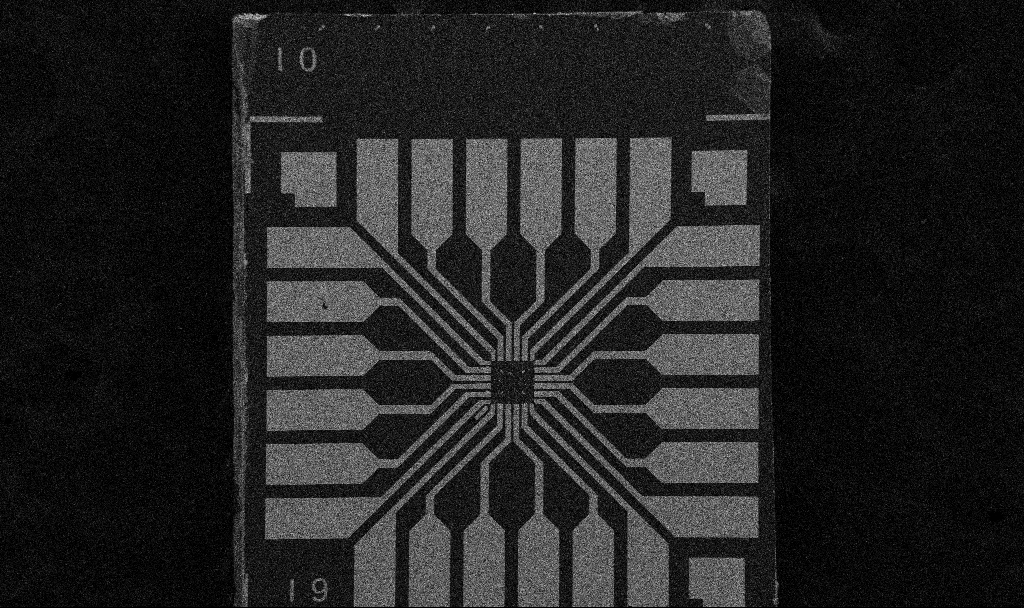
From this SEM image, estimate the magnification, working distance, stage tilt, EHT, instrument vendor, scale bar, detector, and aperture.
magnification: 0.1 K X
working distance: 10.7 mm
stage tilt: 0°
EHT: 5 kV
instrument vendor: Zeiss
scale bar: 200000 nm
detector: SE2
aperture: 30 µm